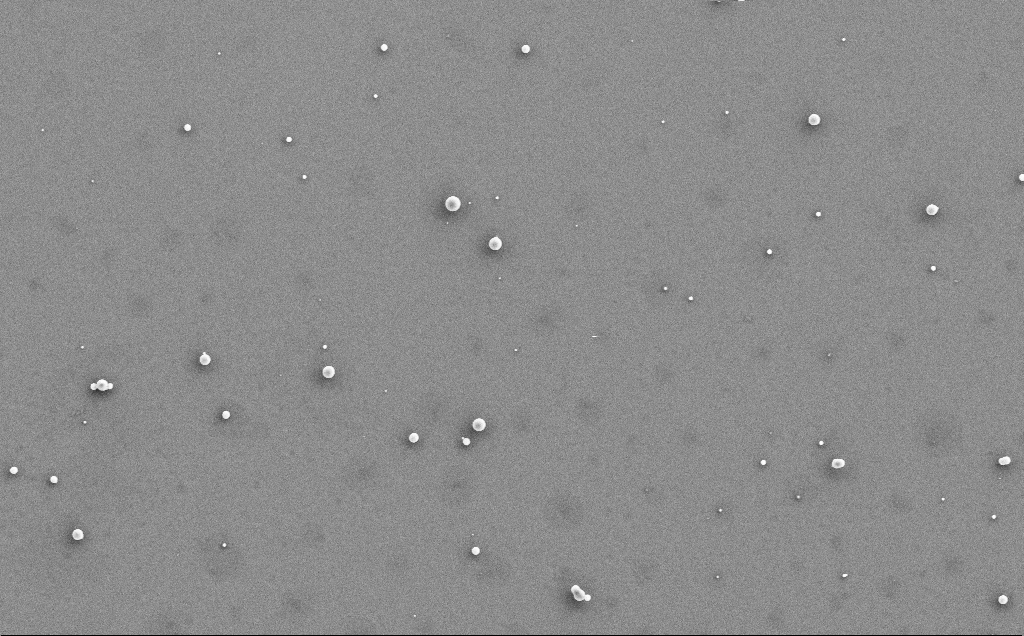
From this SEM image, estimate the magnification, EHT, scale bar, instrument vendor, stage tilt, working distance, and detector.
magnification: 4.01 K X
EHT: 5 kV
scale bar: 10000 nm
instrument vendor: Zeiss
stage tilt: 0°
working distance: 5 mm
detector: SE2